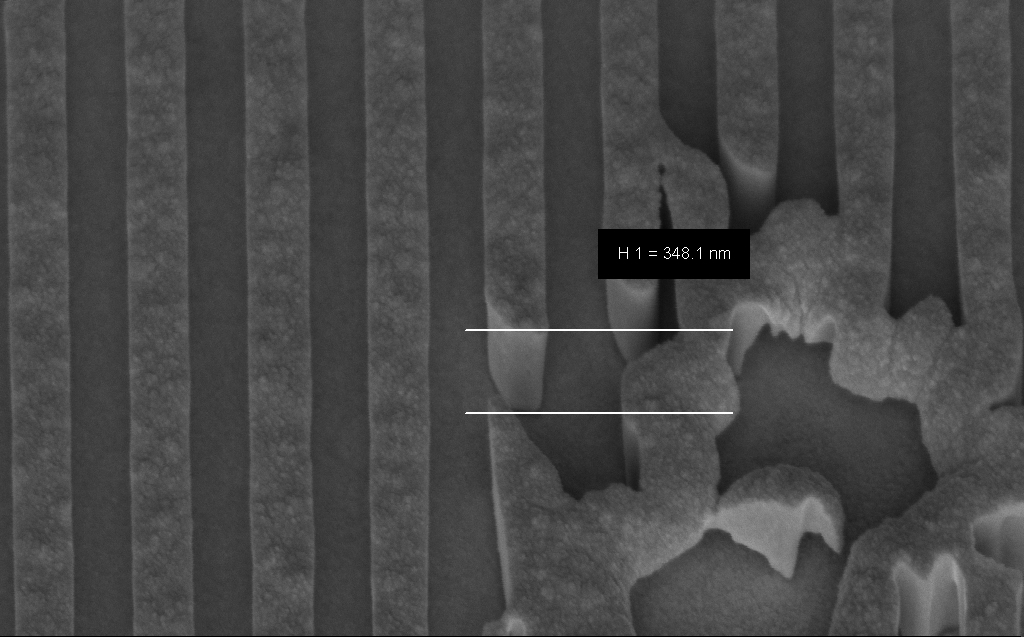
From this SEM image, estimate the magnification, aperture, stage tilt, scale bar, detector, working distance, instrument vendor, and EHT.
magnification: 87.55 K X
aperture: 30 µm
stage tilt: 45°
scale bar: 200 nm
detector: InLens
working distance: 8 mm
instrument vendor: Zeiss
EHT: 5 kV